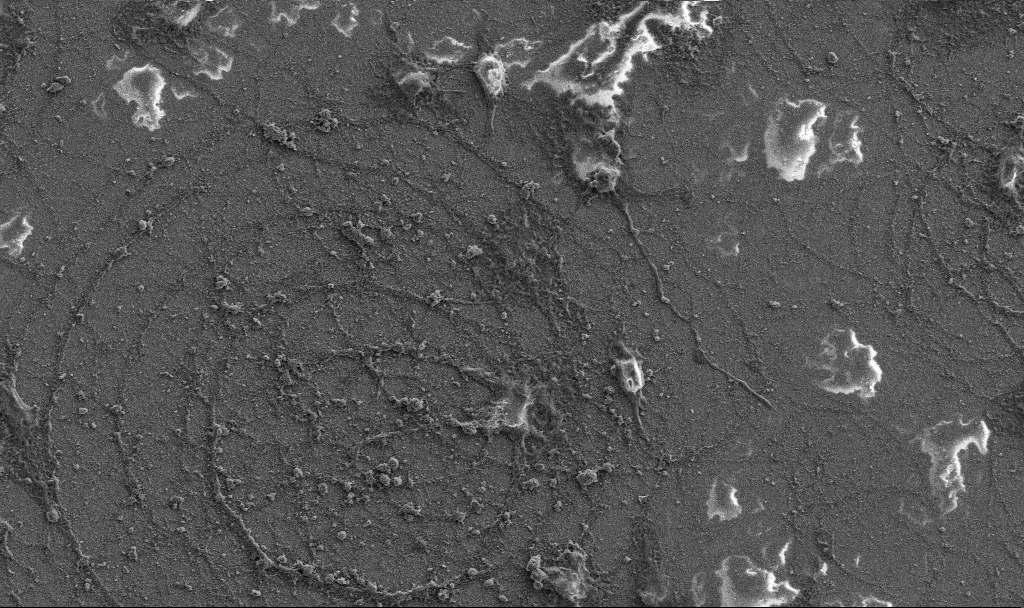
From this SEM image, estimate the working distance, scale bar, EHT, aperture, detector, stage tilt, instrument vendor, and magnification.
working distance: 6.8 mm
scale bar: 20000 nm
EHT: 5 kV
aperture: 30 µm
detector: SE2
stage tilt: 0°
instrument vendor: Zeiss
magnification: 1.5 K X